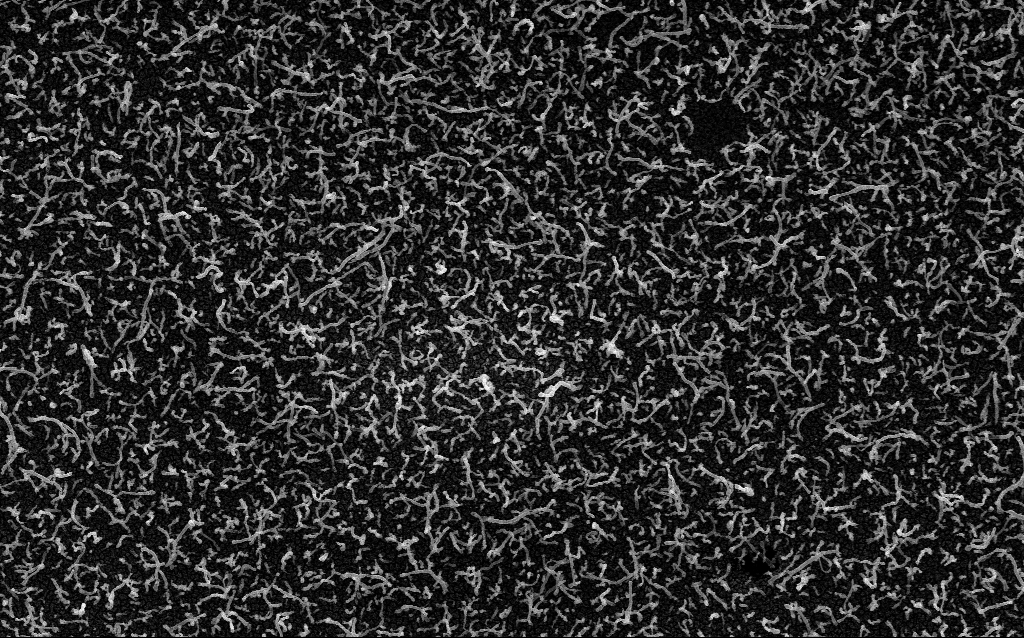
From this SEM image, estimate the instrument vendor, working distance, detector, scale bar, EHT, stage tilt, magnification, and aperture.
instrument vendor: Zeiss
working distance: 2.1 mm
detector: InLens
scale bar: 1000 nm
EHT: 5 kV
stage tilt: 0°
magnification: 20 K X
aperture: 30 µm